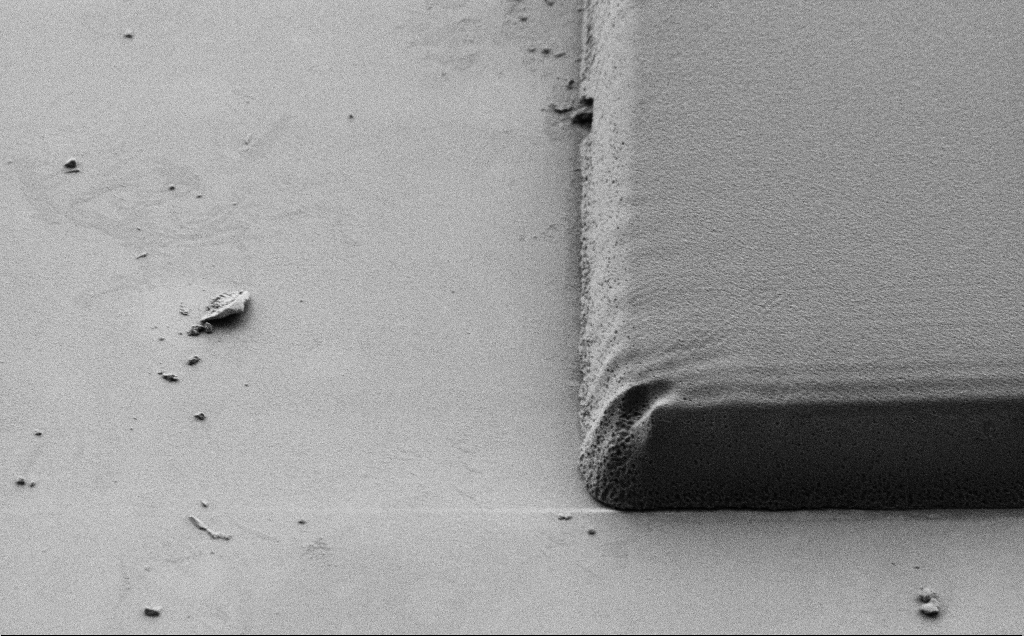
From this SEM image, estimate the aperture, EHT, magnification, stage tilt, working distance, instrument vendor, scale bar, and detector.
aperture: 30 µm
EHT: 1 kV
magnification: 7.54 K X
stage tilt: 45°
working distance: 7 mm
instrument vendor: Zeiss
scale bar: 2000 nm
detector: SE2